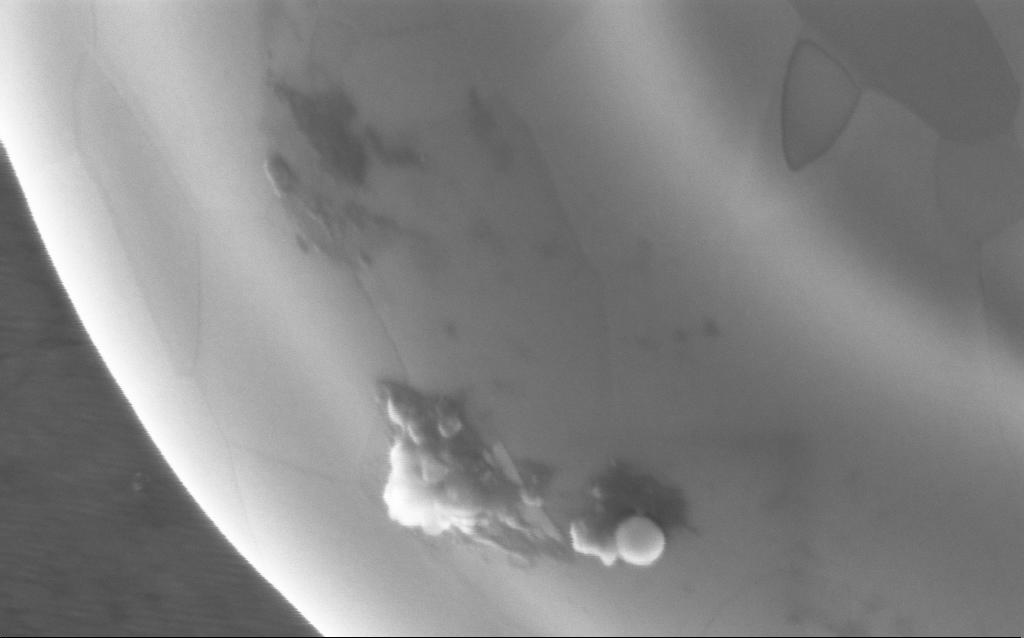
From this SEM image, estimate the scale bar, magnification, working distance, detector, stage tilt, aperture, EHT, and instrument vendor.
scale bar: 200 nm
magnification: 199.88 K X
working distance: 4 mm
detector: InLens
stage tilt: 0°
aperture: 30 µm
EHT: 5 kV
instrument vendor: Zeiss